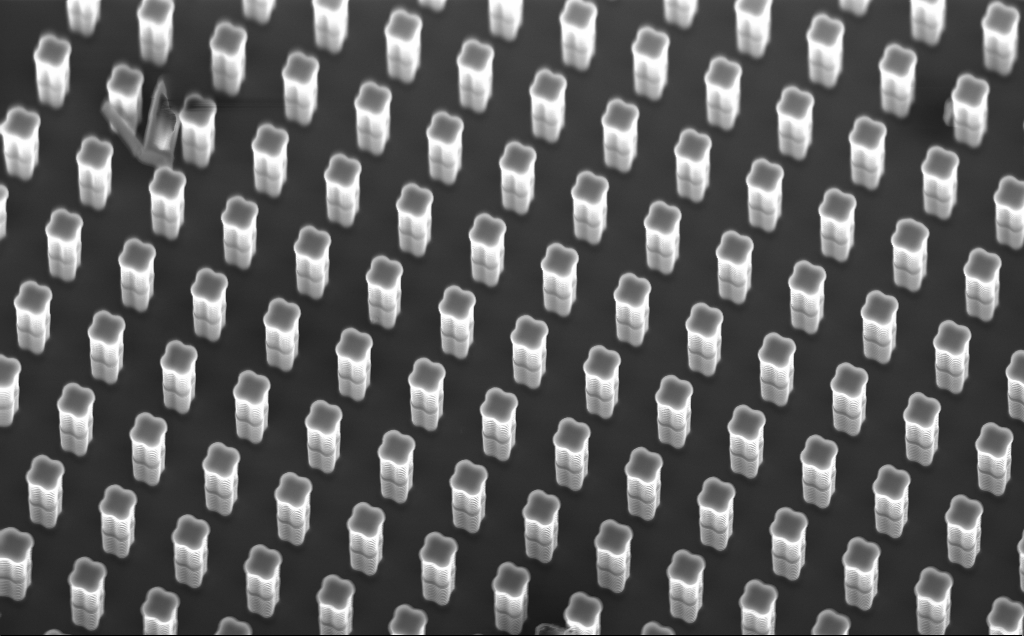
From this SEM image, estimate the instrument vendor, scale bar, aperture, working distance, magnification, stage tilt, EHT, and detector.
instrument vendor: Zeiss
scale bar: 10000 nm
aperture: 120 µm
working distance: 9 mm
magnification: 2.89 K X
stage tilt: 30.3°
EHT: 10 kV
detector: InLens